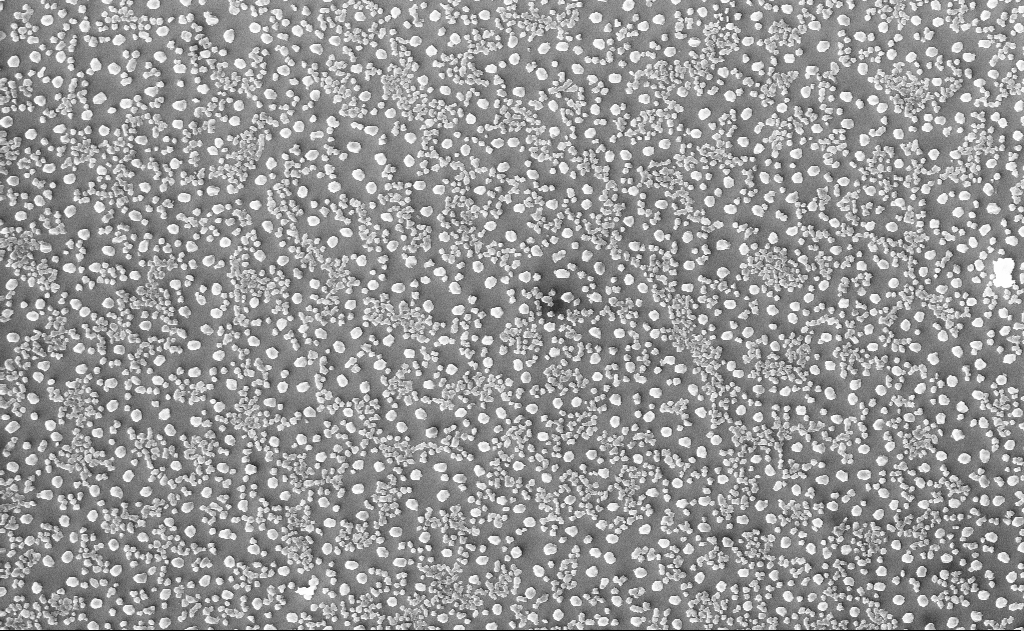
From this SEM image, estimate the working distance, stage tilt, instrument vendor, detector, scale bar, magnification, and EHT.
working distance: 16 mm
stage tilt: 0°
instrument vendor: Zeiss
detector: InLens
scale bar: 2000 nm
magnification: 9.3 K X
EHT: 10 kV